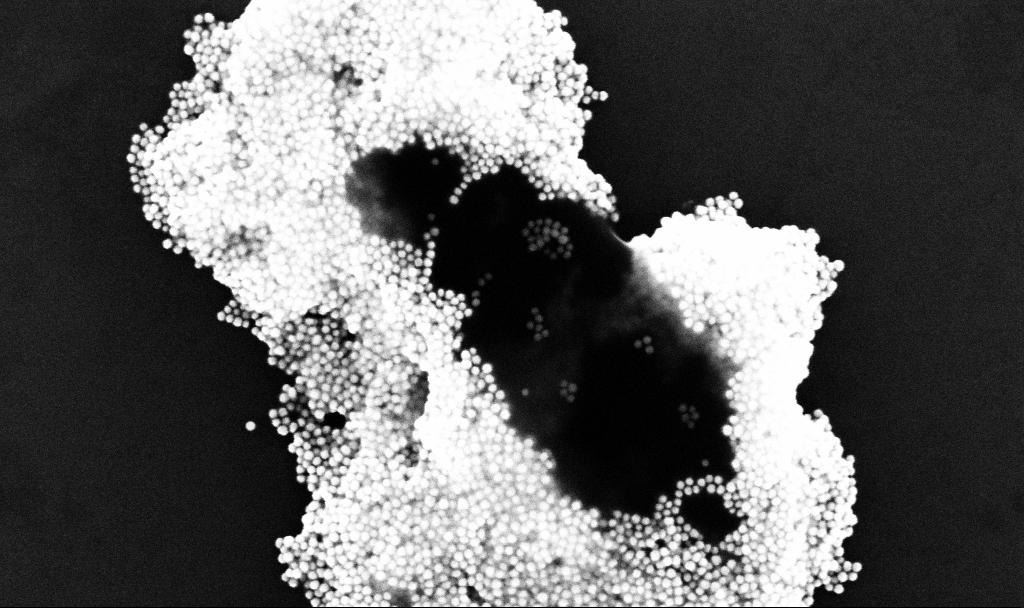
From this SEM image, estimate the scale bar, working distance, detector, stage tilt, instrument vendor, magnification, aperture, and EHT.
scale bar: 200 nm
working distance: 3.3 mm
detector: InLens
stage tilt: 0°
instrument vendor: Zeiss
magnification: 156.58 K X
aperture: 30 µm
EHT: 10 kV